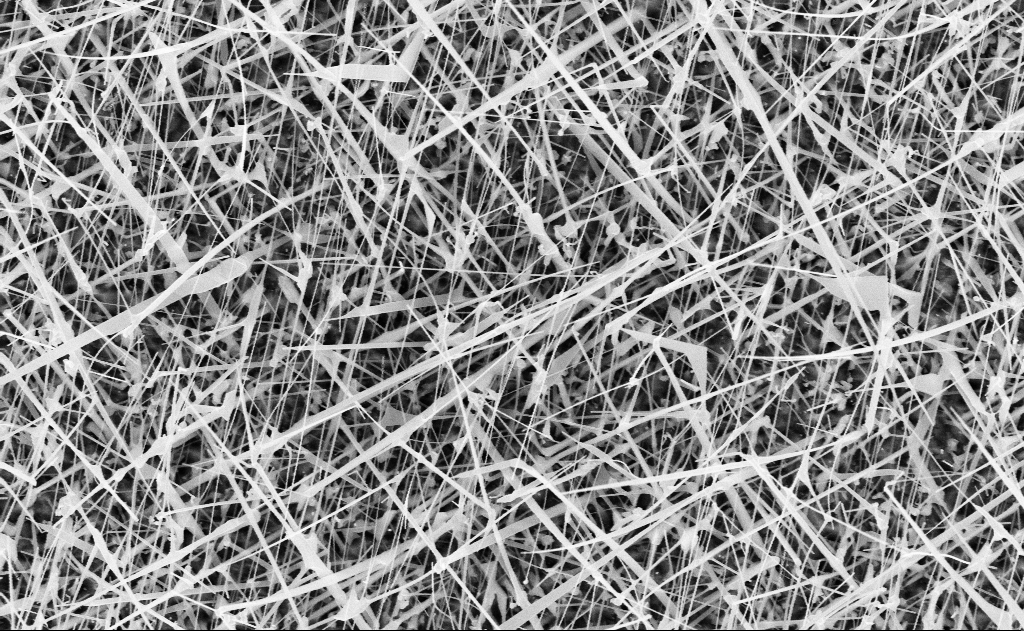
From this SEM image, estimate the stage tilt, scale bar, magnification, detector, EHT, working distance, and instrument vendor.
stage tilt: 0°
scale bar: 1000 nm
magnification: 20 K X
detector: InLens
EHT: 10 kV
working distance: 20 mm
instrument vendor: Zeiss